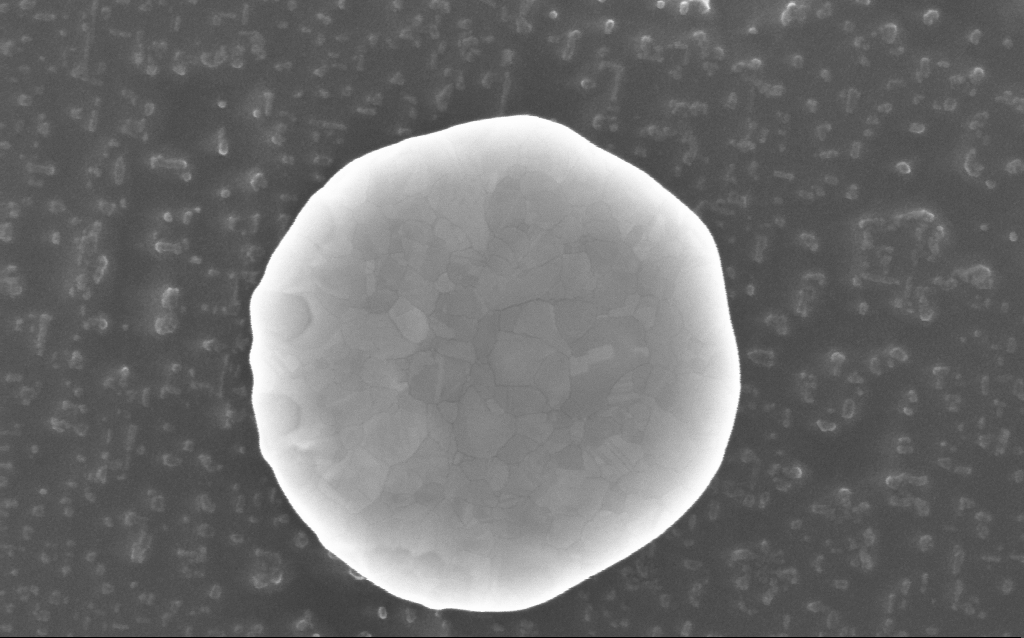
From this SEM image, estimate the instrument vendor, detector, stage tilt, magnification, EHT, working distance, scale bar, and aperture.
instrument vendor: Zeiss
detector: InLens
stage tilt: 0°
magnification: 195.65 K X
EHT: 10 kV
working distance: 5 mm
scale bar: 100 nm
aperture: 30 µm